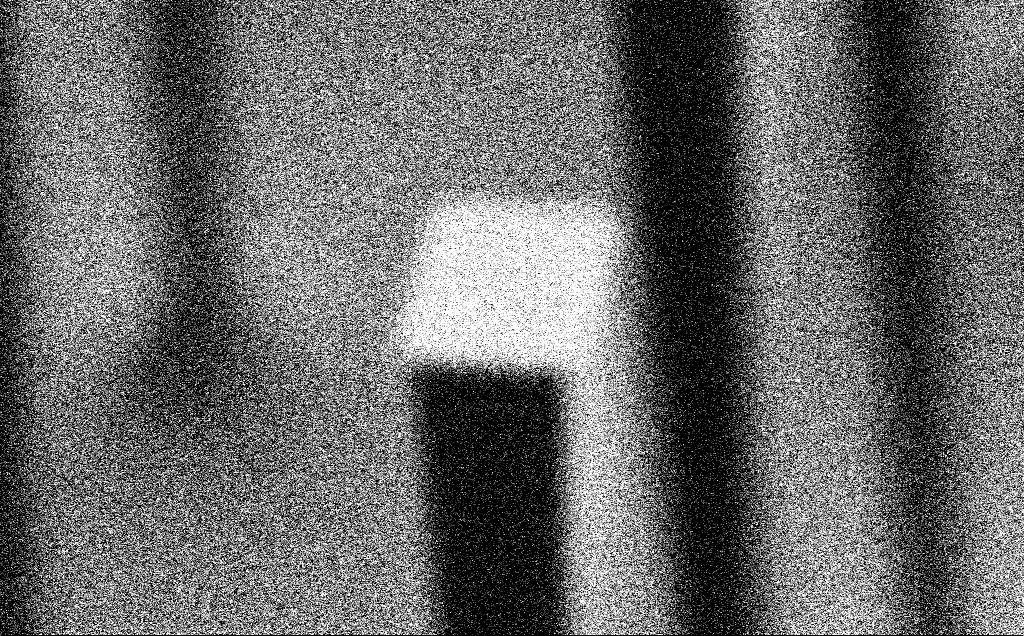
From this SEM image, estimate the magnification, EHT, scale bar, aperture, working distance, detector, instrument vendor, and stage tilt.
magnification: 33.66 K X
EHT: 3 kV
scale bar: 2000 nm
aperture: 30 µm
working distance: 5.2 mm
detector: SE2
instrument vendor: Zeiss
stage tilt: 60°